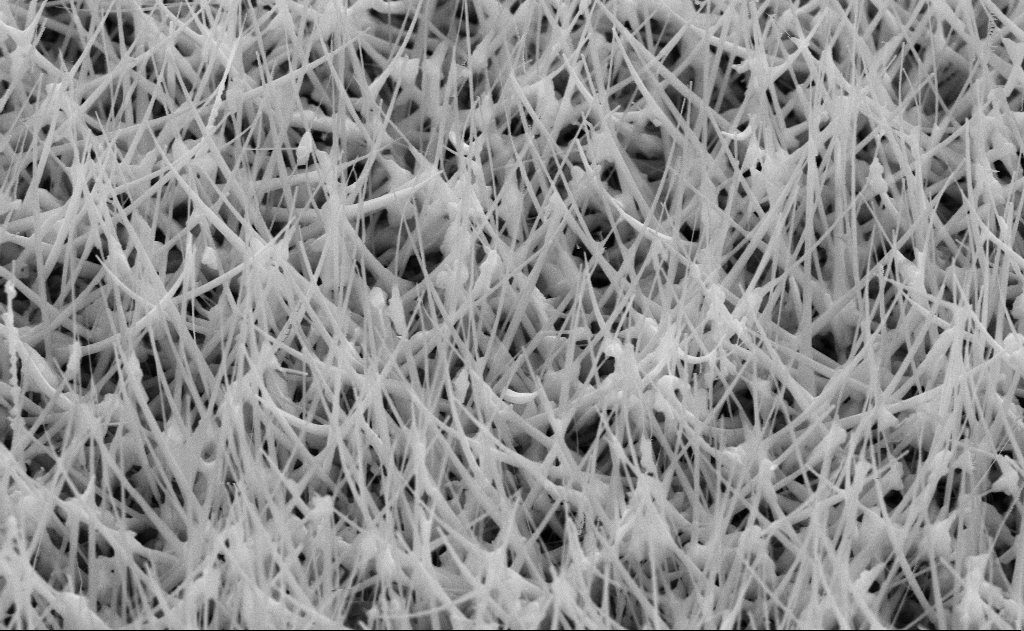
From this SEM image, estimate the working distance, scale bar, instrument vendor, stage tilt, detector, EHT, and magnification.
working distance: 11 mm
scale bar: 1000 nm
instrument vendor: Zeiss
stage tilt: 45°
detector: SE2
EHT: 10 kV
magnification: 40 K X